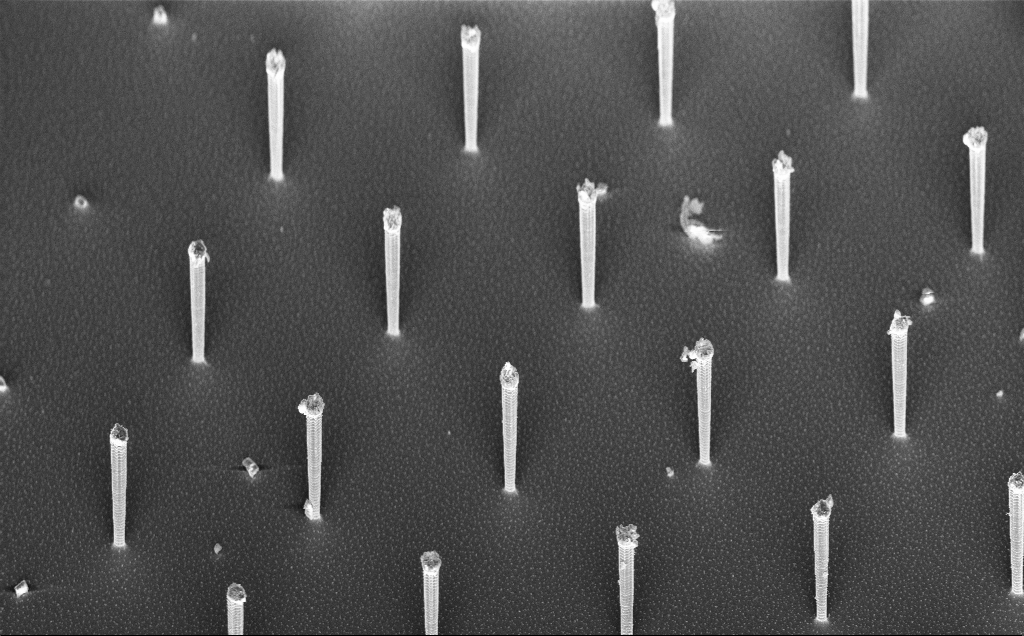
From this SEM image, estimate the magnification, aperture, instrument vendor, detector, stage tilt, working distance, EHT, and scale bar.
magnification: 1.61 K X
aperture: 30 µm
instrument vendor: Zeiss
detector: InLens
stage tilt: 44.9°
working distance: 8 mm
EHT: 7.5 kV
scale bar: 10000 nm